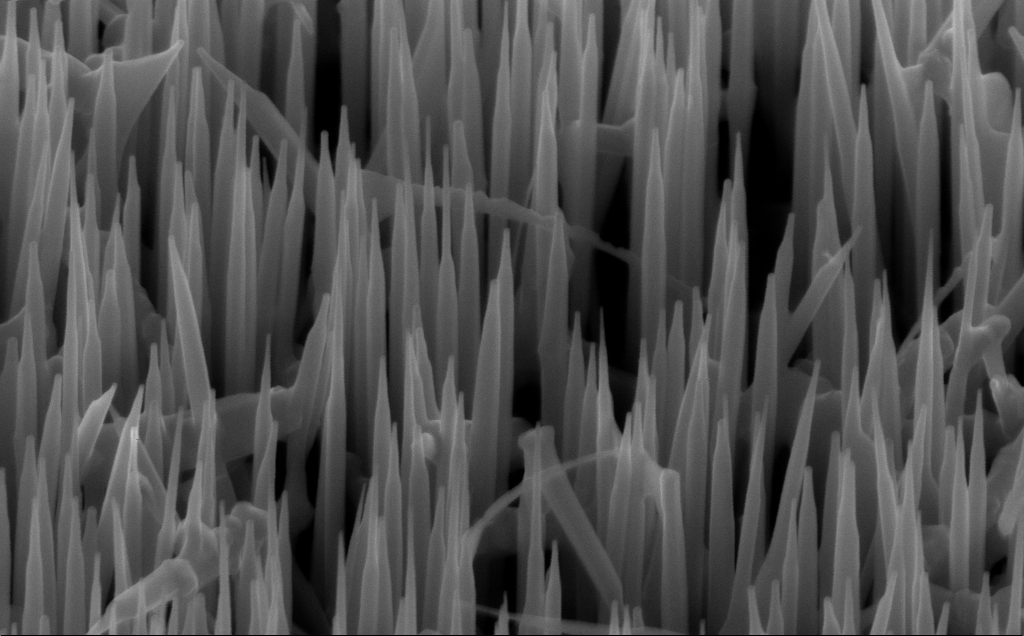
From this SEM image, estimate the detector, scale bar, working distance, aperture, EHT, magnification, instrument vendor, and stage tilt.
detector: InLens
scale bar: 200 nm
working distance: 6 mm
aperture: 30 µm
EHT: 10 kV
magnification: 80 K X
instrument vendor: Zeiss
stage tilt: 45°